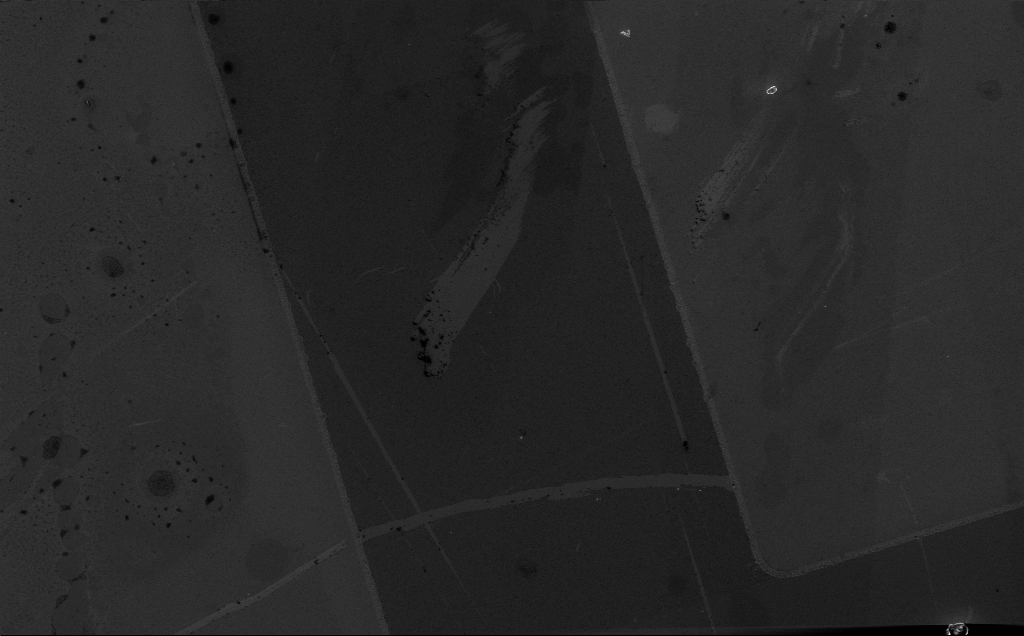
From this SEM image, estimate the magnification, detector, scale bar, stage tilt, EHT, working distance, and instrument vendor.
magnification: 2.4 K X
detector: InLens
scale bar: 10000 nm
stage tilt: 0°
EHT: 5 kV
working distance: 6 mm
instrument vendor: Zeiss